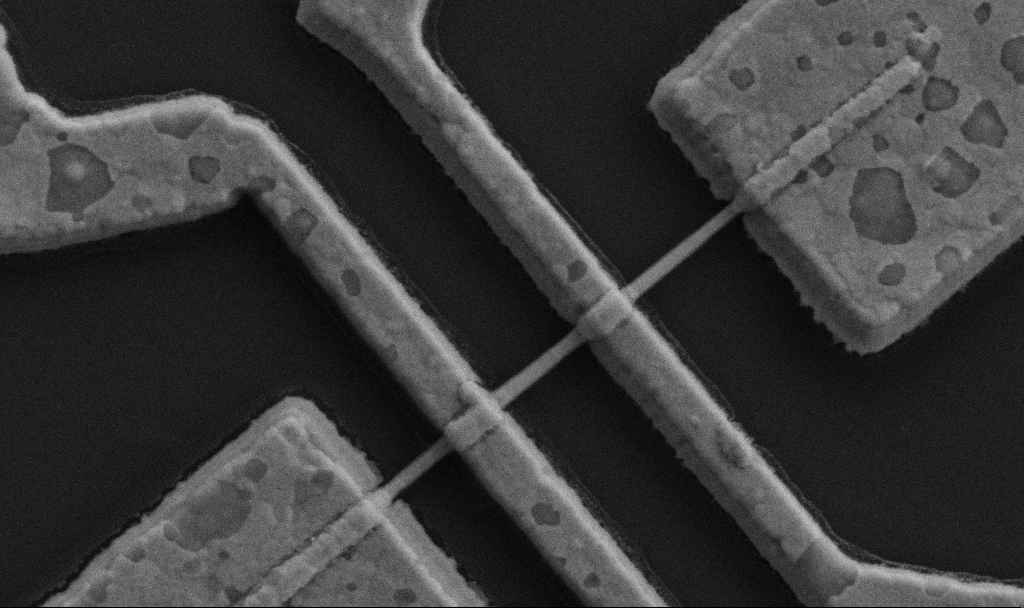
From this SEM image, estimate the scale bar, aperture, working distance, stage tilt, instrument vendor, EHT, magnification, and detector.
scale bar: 1000 nm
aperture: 30 µm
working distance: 9.7 mm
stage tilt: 0°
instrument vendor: Zeiss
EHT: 5 kV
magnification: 60 K X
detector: SE2